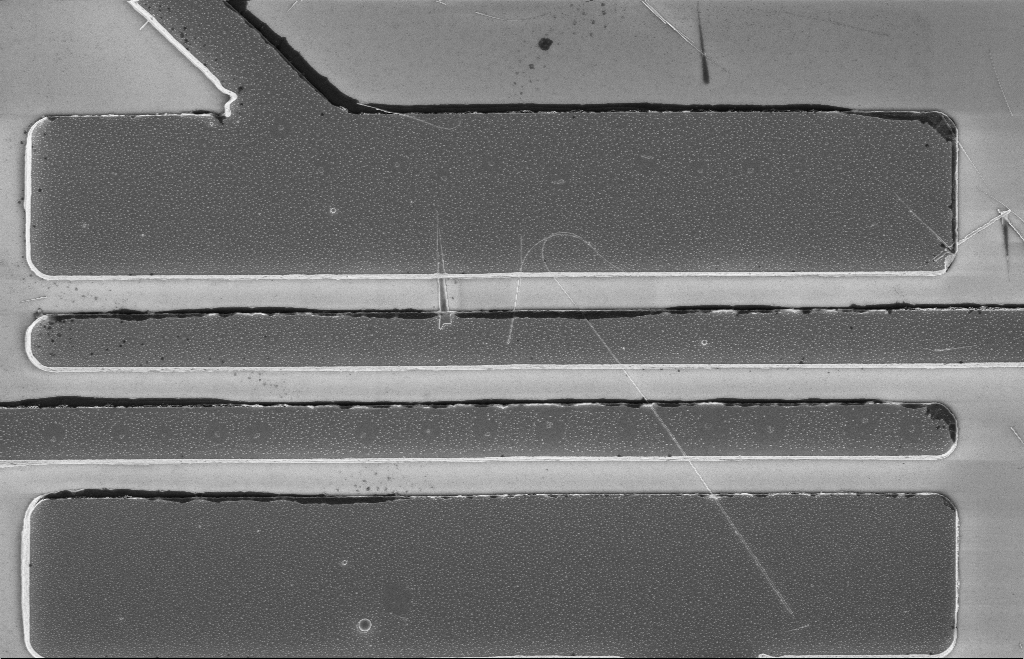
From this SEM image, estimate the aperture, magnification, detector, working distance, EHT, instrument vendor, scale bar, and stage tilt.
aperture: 20 µm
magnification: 5.65 K X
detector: InLens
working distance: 12 mm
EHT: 5 kV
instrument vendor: Zeiss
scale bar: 2000 nm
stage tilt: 0°